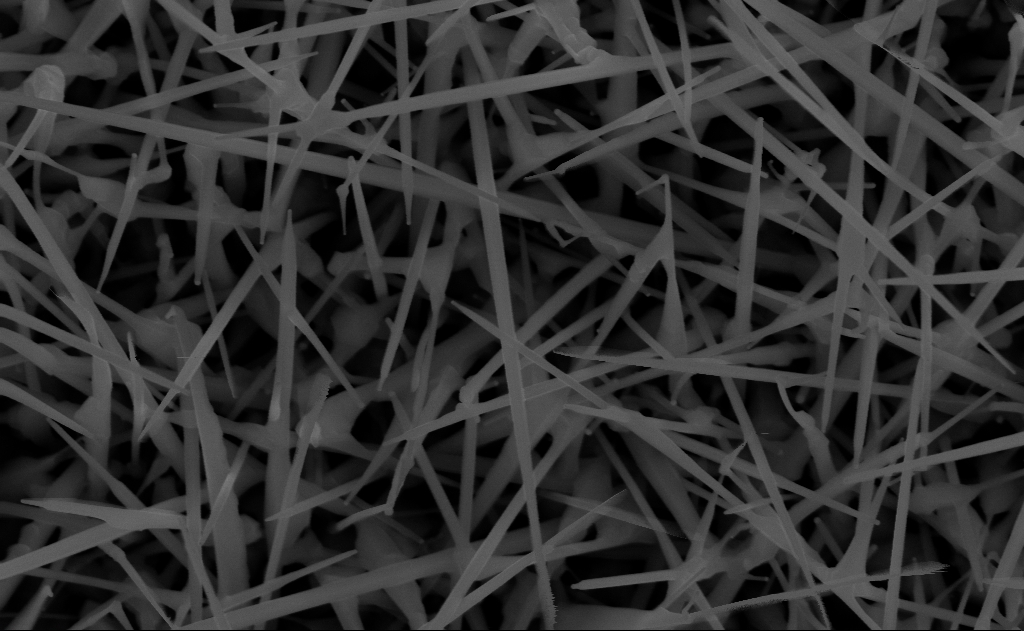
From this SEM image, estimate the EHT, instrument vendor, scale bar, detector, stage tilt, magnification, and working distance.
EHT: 10 kV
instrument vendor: Zeiss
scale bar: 1000 nm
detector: InLens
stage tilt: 0°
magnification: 60 K X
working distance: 10 mm